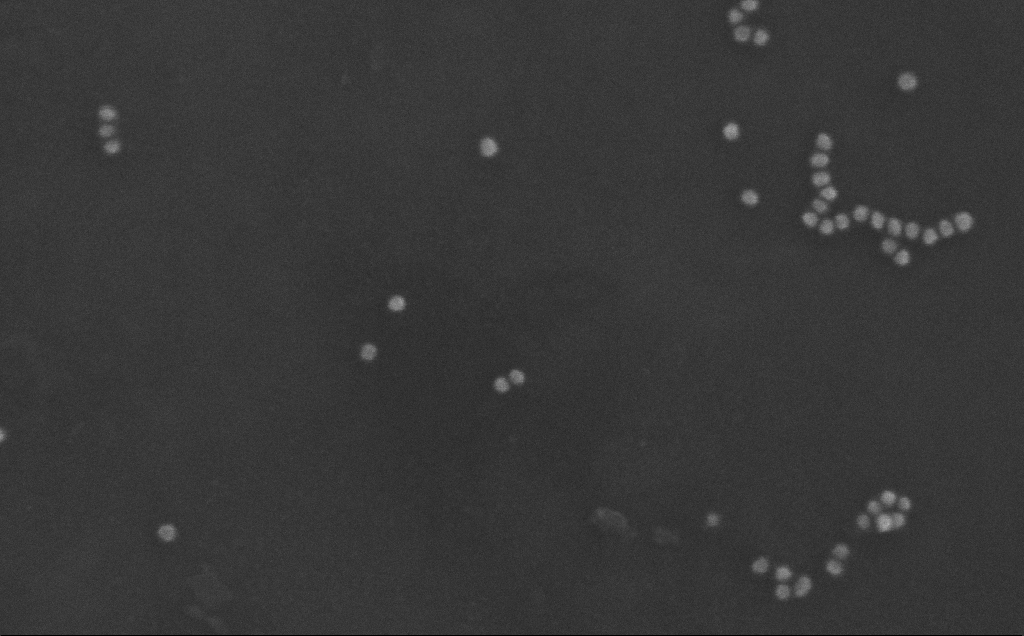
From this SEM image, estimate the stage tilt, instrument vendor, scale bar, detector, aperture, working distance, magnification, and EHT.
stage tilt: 0°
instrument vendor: Zeiss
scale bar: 200 nm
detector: InLens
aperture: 30 µm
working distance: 3 mm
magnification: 354.33 K X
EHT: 10 kV